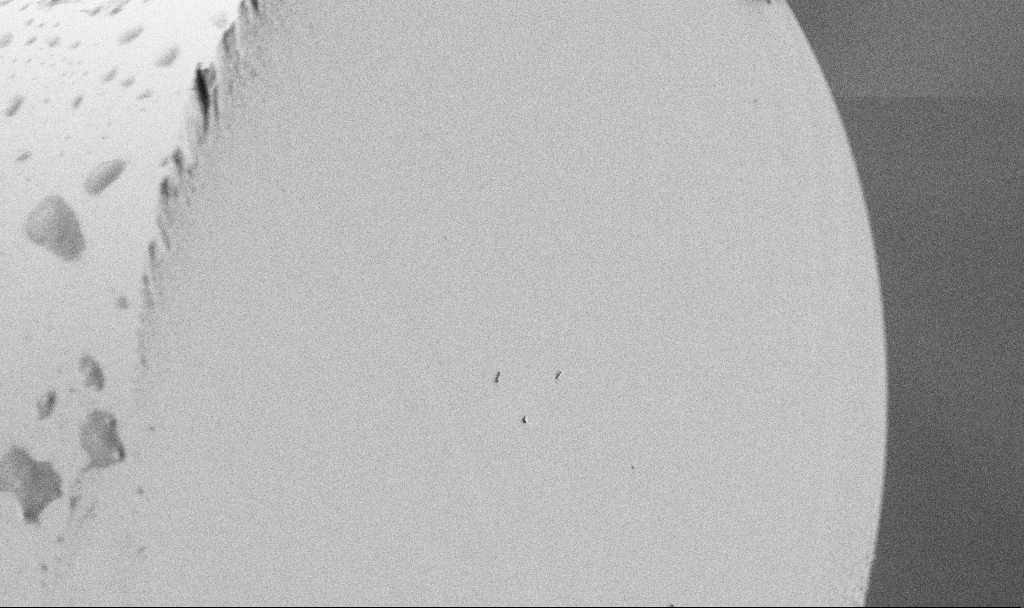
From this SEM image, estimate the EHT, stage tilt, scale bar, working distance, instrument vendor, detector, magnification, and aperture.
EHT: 1.5 kV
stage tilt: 45°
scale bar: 10000 nm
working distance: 3.4 mm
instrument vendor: Zeiss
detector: SE2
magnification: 3.39 K X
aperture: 30 µm